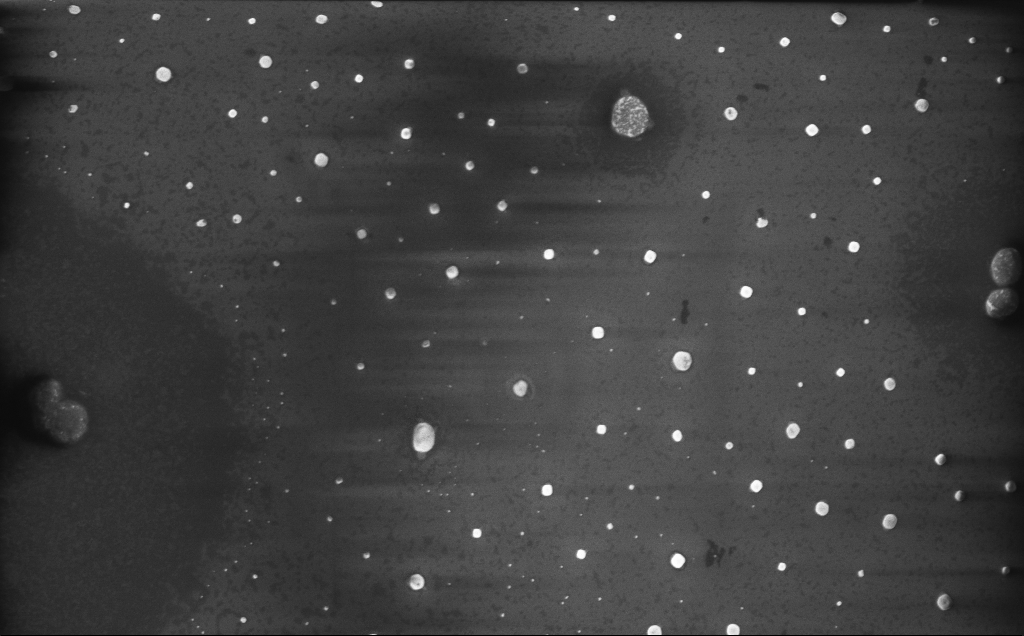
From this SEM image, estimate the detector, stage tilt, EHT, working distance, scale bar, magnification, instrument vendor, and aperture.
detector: InLens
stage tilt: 0°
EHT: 10 kV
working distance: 5 mm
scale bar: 1000 nm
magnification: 20 K X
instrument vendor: Zeiss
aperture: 30 µm